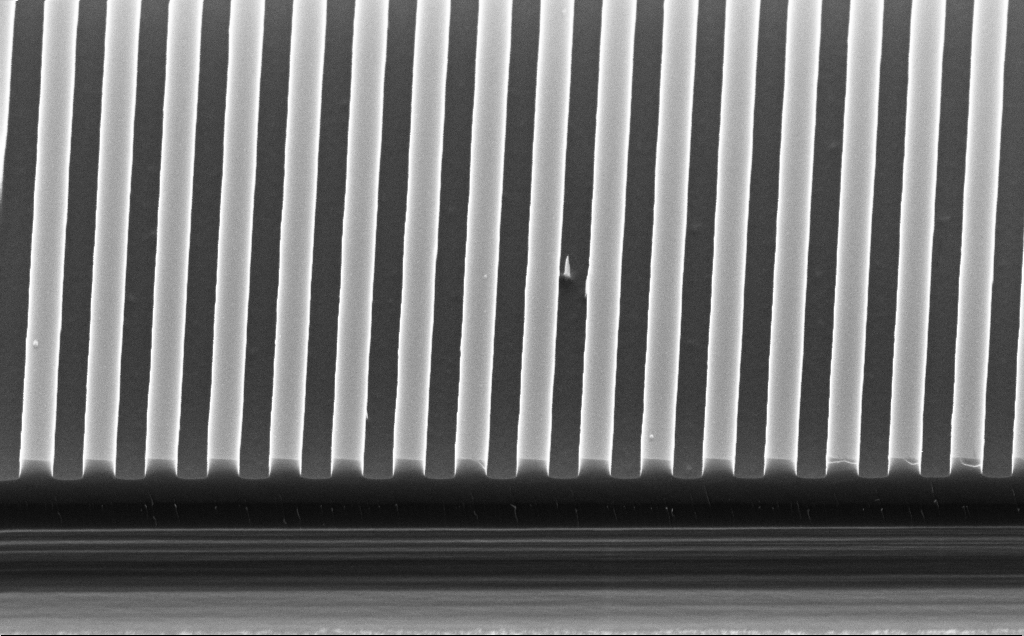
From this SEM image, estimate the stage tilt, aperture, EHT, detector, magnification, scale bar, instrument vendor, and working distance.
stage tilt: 45°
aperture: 30 µm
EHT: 10 kV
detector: InLens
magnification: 45.85 K X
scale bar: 1000 nm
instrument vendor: Zeiss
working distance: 4 mm